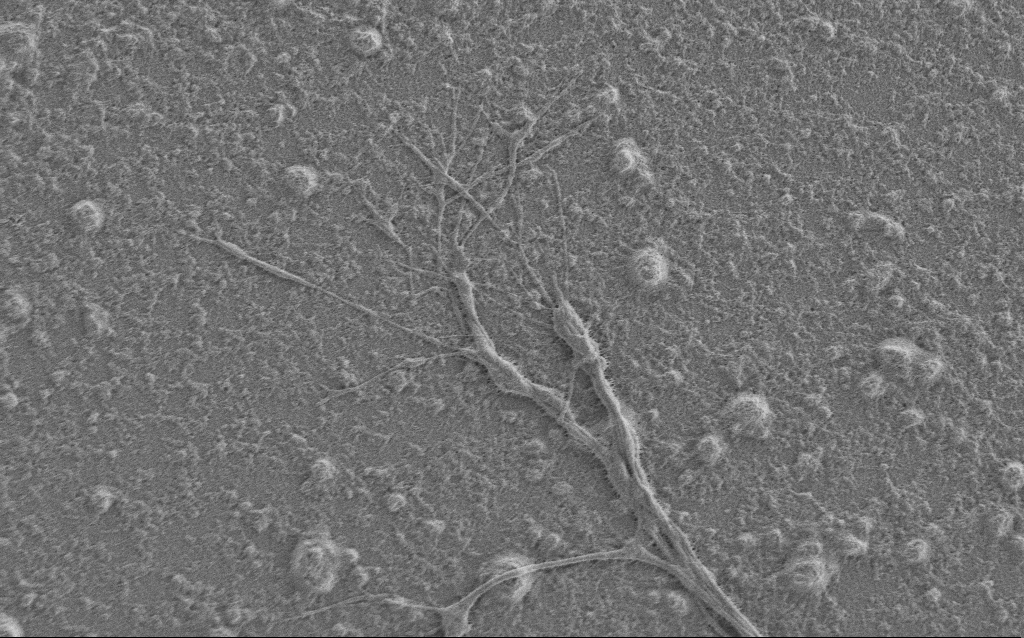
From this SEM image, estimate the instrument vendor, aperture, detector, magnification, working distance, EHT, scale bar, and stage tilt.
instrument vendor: Zeiss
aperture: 30 µm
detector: SE2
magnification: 6 K X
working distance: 6 mm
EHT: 1 kV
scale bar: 10000 nm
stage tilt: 0°